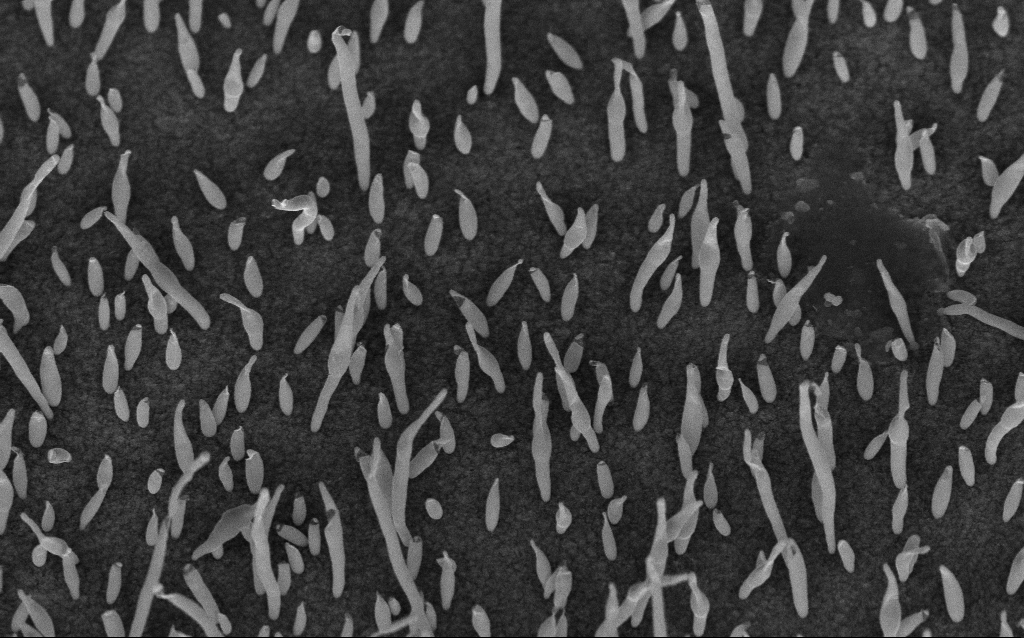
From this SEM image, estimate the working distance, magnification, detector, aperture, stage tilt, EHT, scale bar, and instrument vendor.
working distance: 7 mm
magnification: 50 K X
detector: InLens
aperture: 30 µm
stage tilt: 45°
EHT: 5 kV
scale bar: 1000 nm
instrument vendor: Zeiss